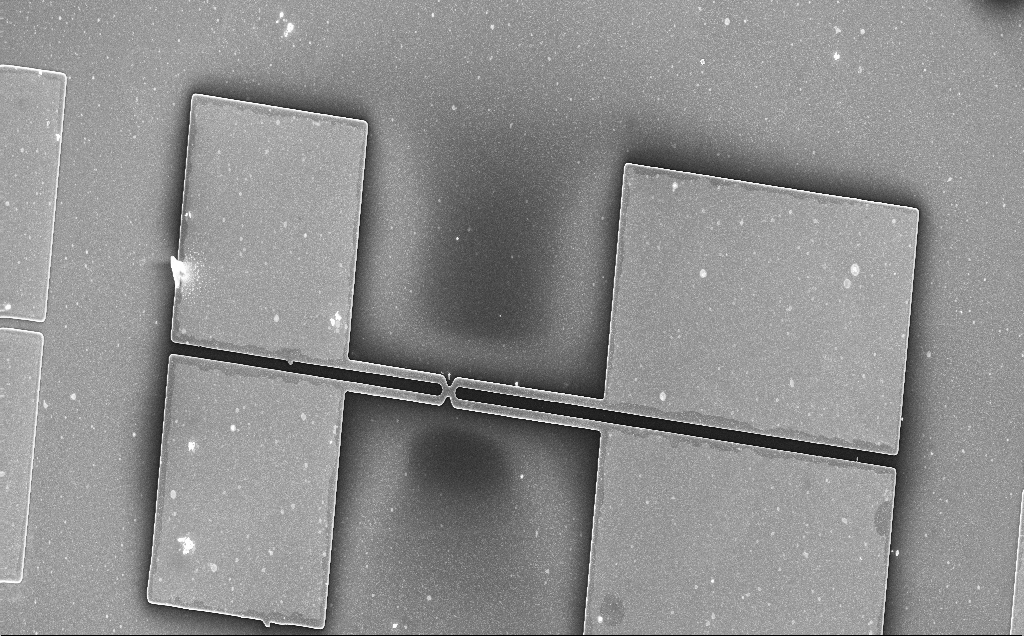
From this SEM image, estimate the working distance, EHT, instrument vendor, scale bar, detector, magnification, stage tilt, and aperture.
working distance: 4 mm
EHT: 15 kV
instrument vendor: Zeiss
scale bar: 100000 nm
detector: InLens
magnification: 0.32 K X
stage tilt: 0°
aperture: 30 µm